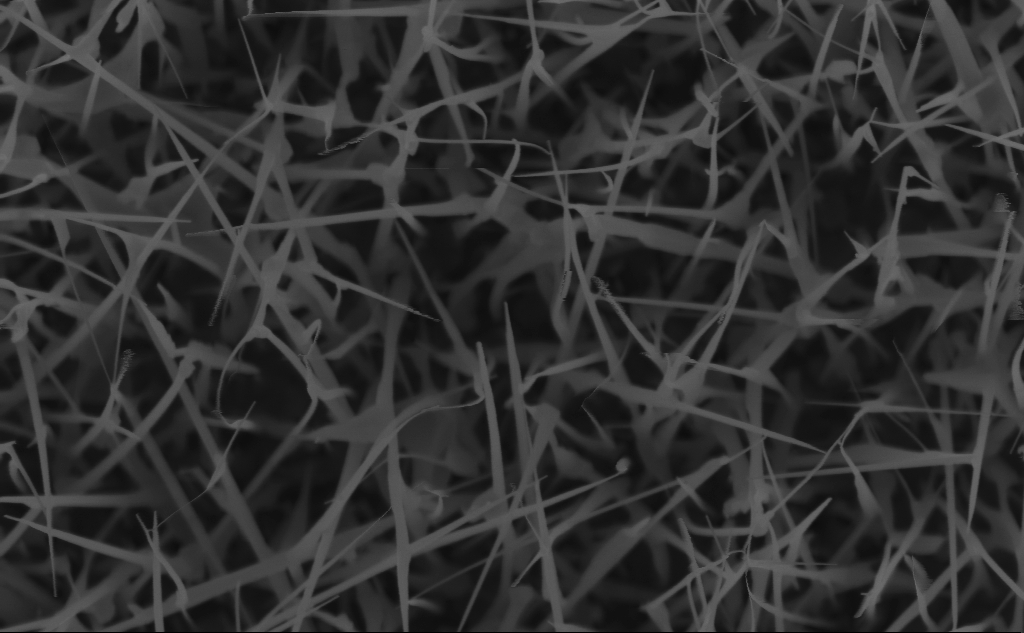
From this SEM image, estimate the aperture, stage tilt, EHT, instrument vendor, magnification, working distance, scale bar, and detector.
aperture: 30 µm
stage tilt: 0°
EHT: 5 kV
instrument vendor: Zeiss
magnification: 62.12 K X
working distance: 3 mm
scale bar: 1000 nm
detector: InLens